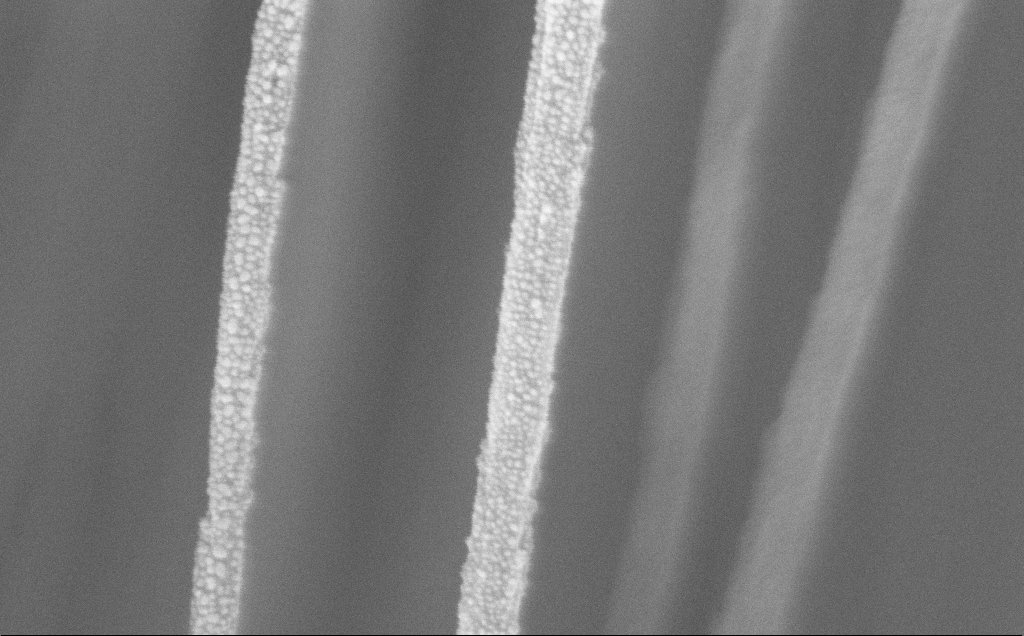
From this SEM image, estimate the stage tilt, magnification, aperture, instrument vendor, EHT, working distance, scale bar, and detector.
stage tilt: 0°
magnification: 163.96 K X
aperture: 30 µm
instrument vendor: Zeiss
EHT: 5 kV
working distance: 11 mm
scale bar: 200 nm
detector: InLens